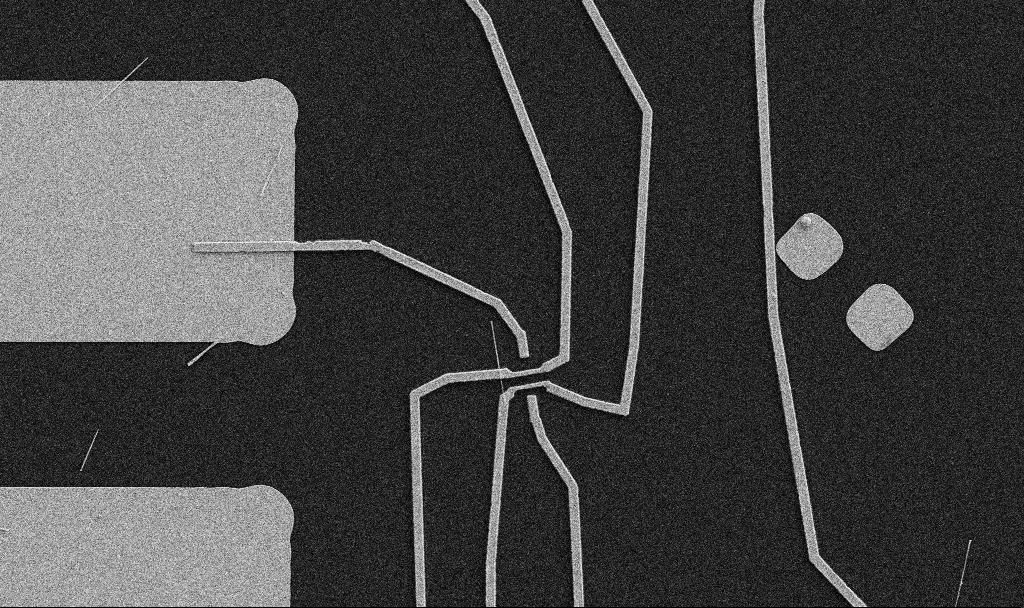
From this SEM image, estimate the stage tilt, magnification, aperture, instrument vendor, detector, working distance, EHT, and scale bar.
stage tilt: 0°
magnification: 5 K X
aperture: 30 µm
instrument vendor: Zeiss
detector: SE2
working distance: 10.7 mm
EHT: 5 kV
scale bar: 10000 nm